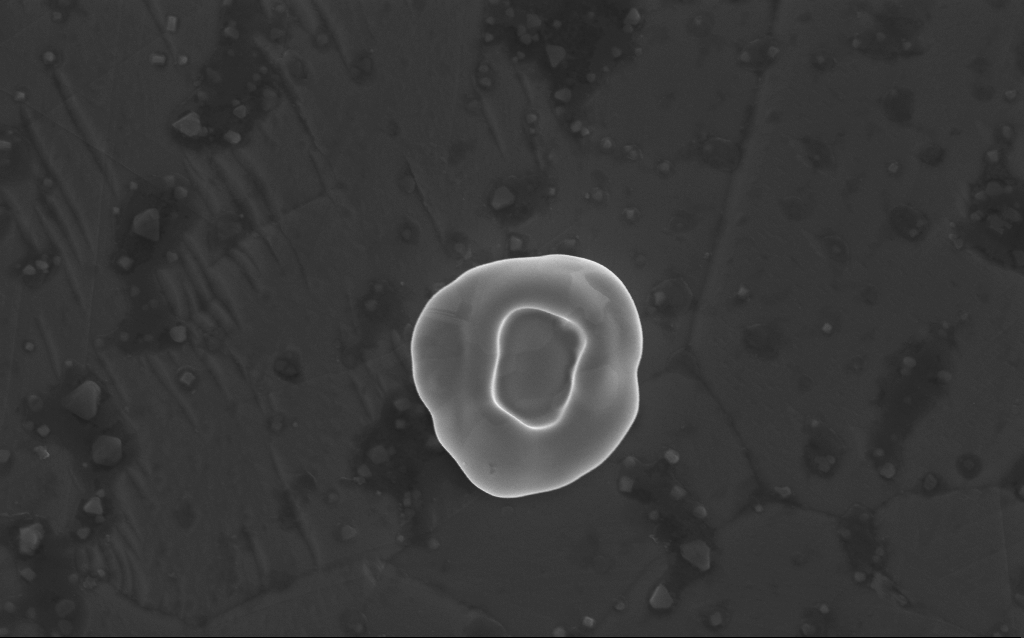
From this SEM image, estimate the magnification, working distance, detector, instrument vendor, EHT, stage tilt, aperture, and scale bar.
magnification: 62.87 K X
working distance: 4 mm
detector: InLens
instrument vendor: Zeiss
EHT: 5 kV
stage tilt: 0°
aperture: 30 µm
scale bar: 1000 nm